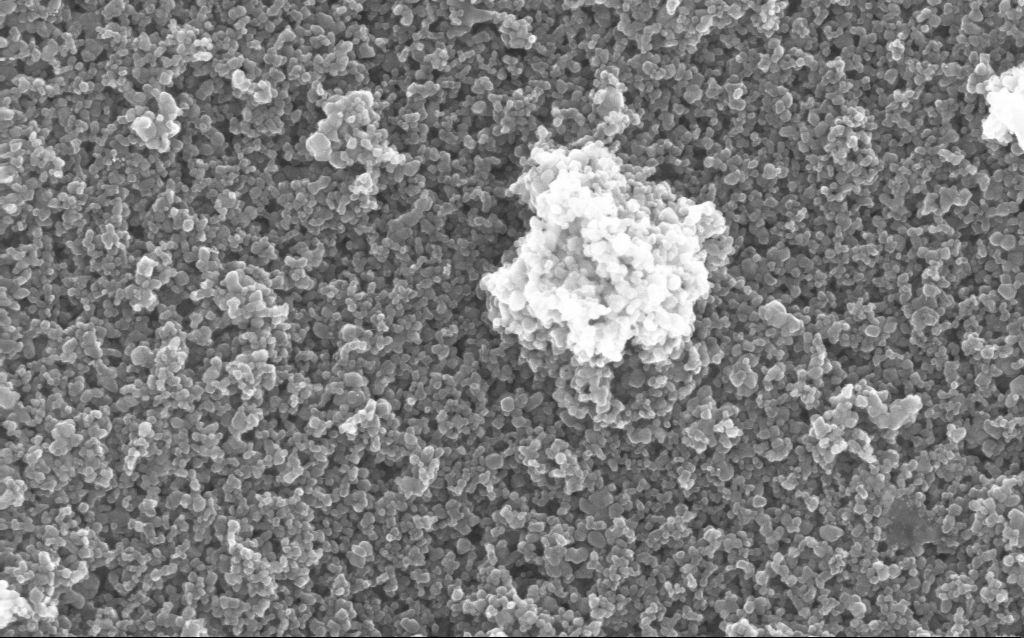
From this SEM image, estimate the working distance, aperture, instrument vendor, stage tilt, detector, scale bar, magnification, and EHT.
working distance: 4.2 mm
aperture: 30 µm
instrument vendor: Zeiss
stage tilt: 0°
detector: InLens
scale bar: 200 nm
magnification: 130 K X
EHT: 10 kV